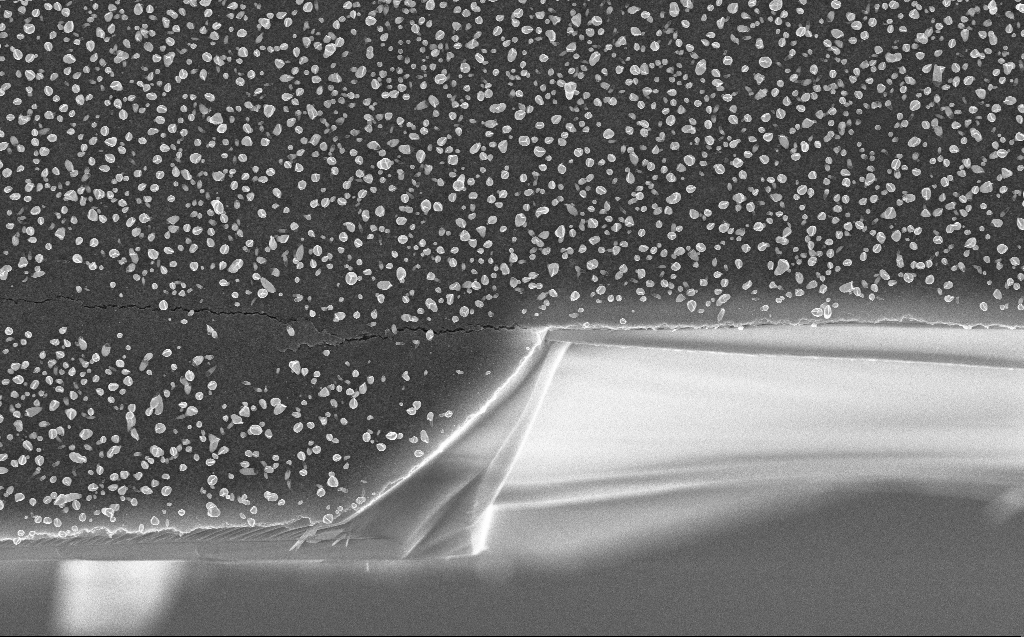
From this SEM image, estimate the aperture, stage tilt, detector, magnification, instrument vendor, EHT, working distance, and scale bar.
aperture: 30 µm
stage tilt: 0°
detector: InLens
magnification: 18.79 K X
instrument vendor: Zeiss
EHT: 10 kV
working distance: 3 mm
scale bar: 2000 nm